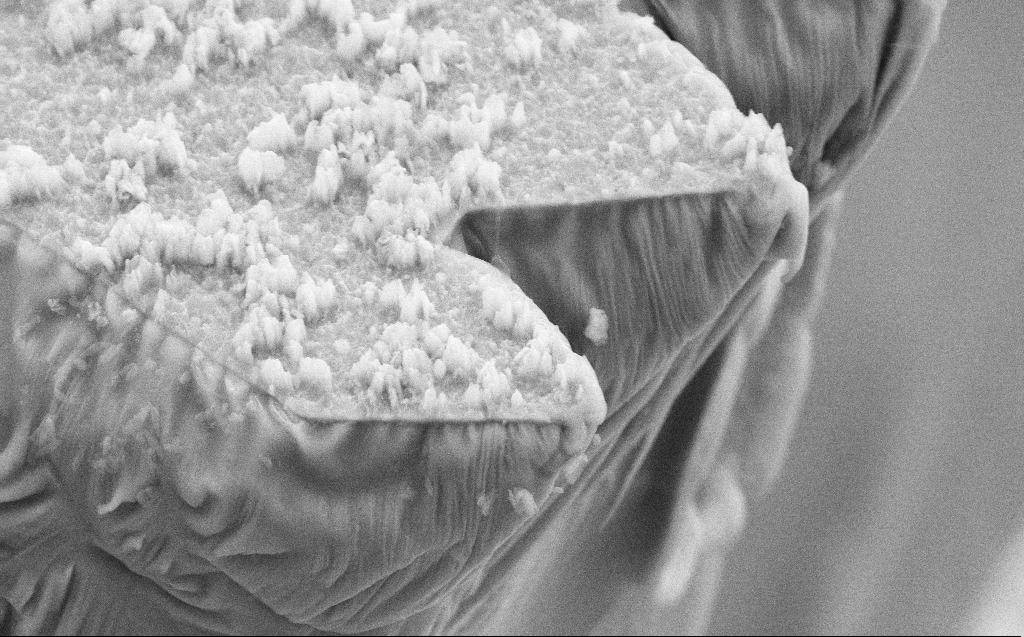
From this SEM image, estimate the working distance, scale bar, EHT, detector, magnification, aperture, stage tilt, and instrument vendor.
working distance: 8 mm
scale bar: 2000 nm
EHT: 20 kV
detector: SE2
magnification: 9.62 K X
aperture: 30 µm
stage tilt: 45°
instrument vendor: Zeiss